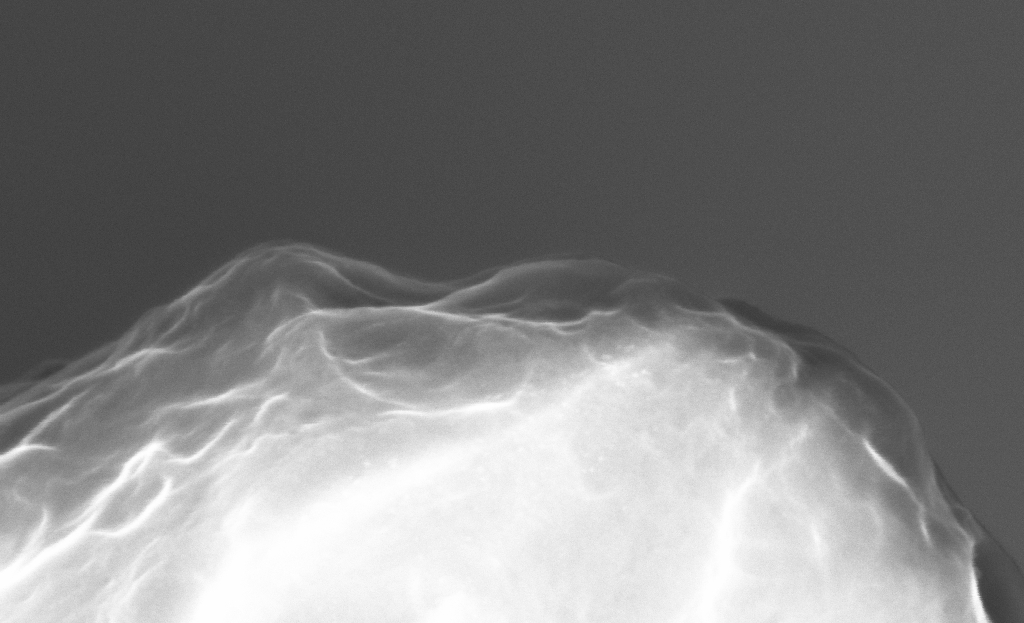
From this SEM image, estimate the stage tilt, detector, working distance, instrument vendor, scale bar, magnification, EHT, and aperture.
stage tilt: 40°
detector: InLens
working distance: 5 mm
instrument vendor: Zeiss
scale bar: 200 nm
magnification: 303.08 K X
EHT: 10 kV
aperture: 30 µm